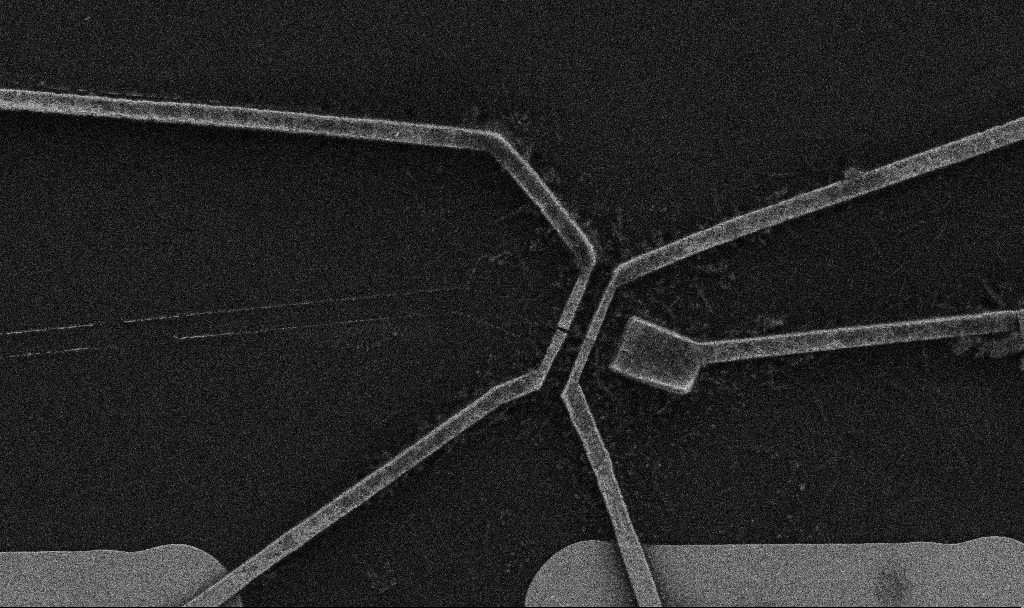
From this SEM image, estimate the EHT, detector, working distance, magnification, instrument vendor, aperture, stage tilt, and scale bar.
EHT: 5 kV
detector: SE2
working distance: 9.7 mm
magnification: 10 K X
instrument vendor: Zeiss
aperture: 30 µm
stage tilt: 0°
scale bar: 2000 nm